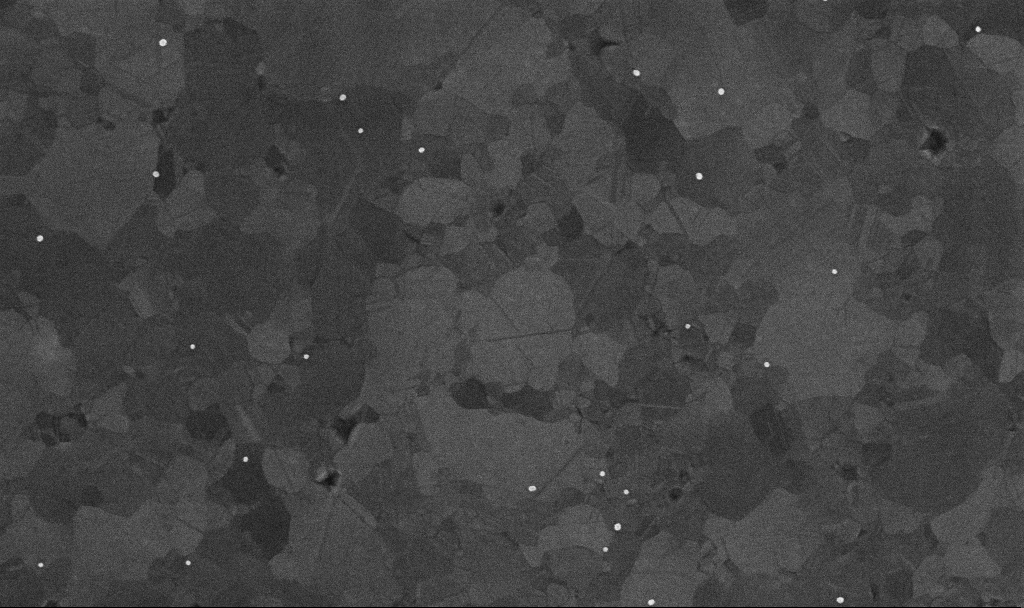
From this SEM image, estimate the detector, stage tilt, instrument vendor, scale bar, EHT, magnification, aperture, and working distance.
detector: InLens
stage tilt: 0°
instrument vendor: Zeiss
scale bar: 200 nm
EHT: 10 kV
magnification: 100 K X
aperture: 30 µm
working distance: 3.3 mm